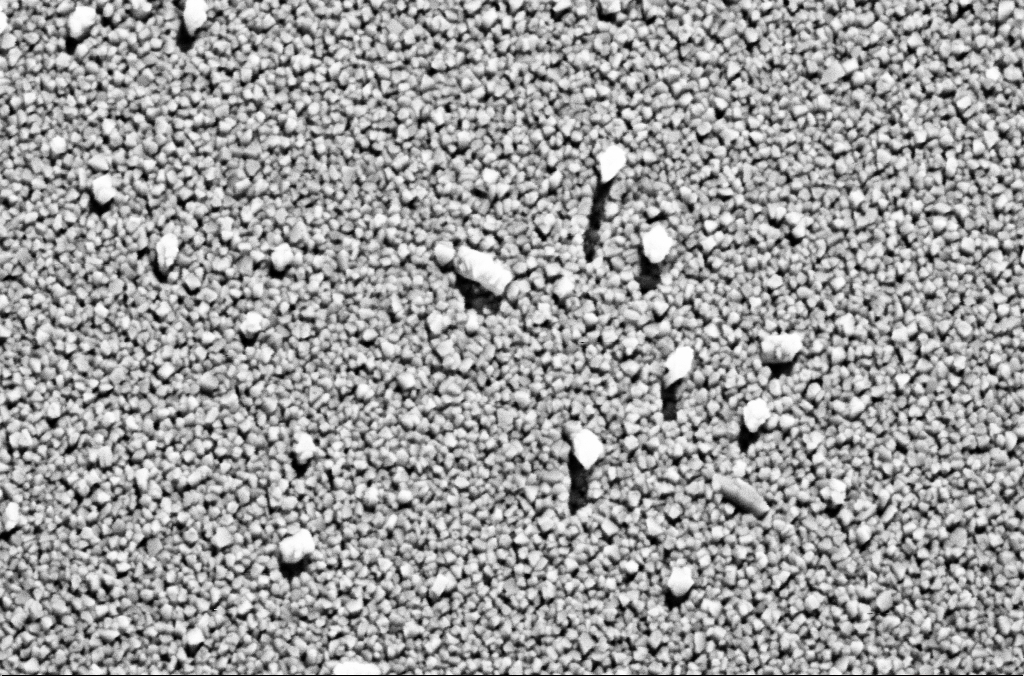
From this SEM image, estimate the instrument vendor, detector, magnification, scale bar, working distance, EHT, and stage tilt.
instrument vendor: Zeiss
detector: SE2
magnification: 146.44 K X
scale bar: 100 nm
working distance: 8.5 mm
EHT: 5 kV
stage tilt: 0°